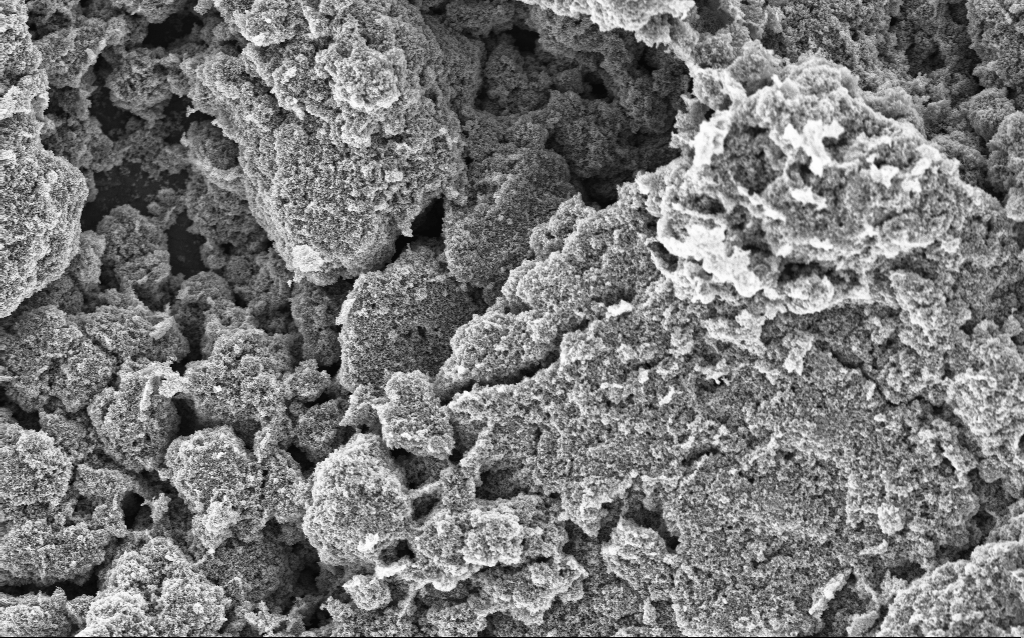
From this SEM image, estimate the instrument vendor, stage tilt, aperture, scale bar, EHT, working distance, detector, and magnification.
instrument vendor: Zeiss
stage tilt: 0°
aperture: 30 µm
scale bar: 10000 nm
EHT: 5 kV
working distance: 4.4 mm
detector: InLens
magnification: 6.42 K X